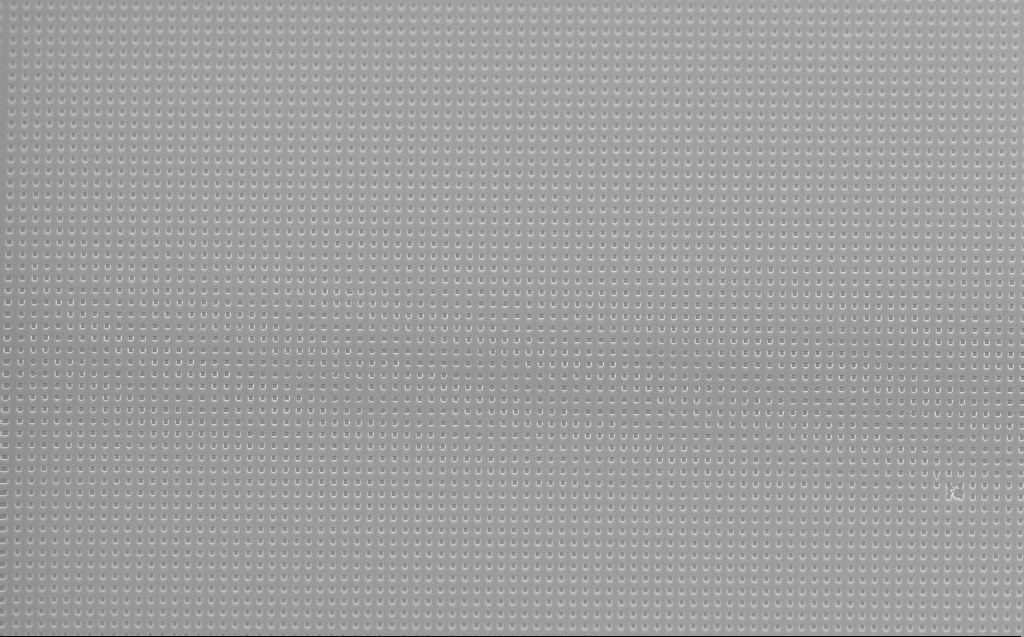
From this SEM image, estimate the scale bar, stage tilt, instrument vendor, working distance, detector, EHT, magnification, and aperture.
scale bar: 2000 nm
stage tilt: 45°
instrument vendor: Zeiss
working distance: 5 mm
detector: InLens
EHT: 10 kV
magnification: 9 K X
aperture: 30 µm